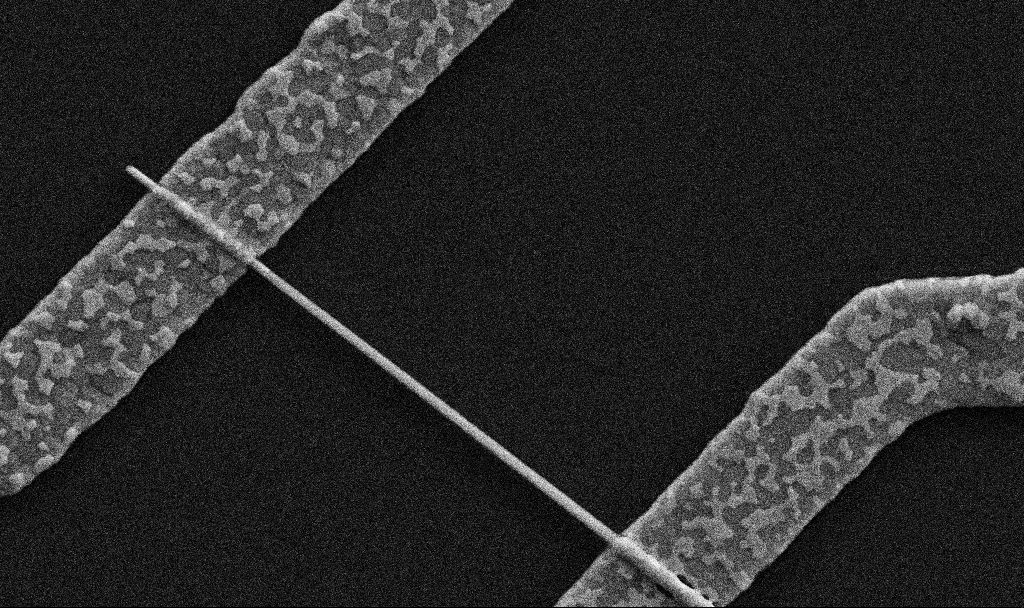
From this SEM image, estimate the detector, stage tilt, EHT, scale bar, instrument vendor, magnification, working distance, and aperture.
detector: SE2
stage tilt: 0°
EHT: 5 kV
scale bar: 1000 nm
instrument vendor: Zeiss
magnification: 51.34 K X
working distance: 6.7 mm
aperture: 30 µm